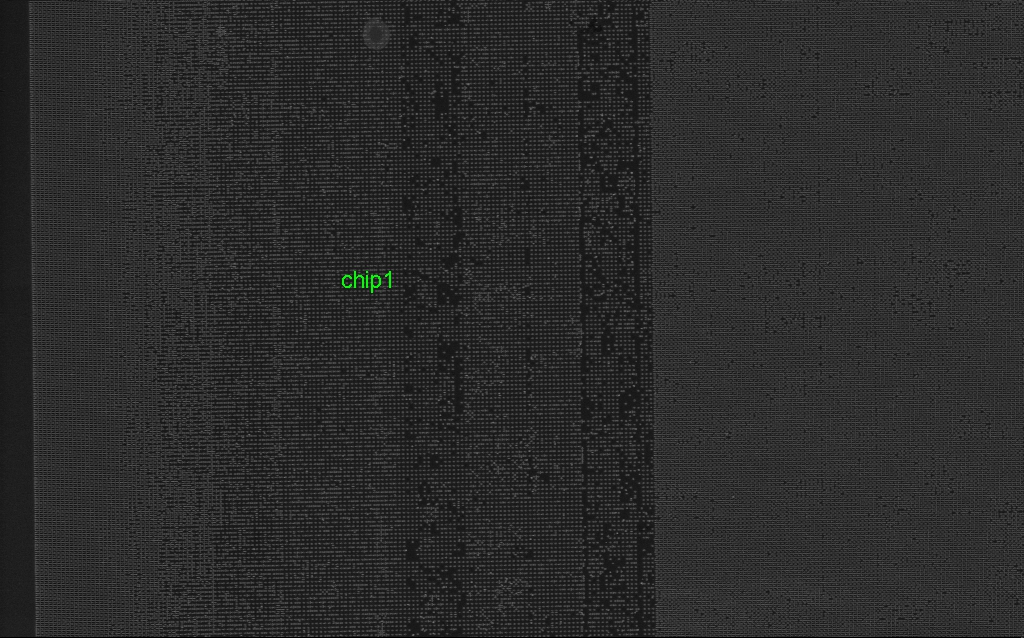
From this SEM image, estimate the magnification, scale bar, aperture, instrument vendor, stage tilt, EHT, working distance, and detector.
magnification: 3.38 K X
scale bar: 10000 nm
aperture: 30 µm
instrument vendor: Zeiss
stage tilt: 0°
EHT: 5 kV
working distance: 4.3 mm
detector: InLens